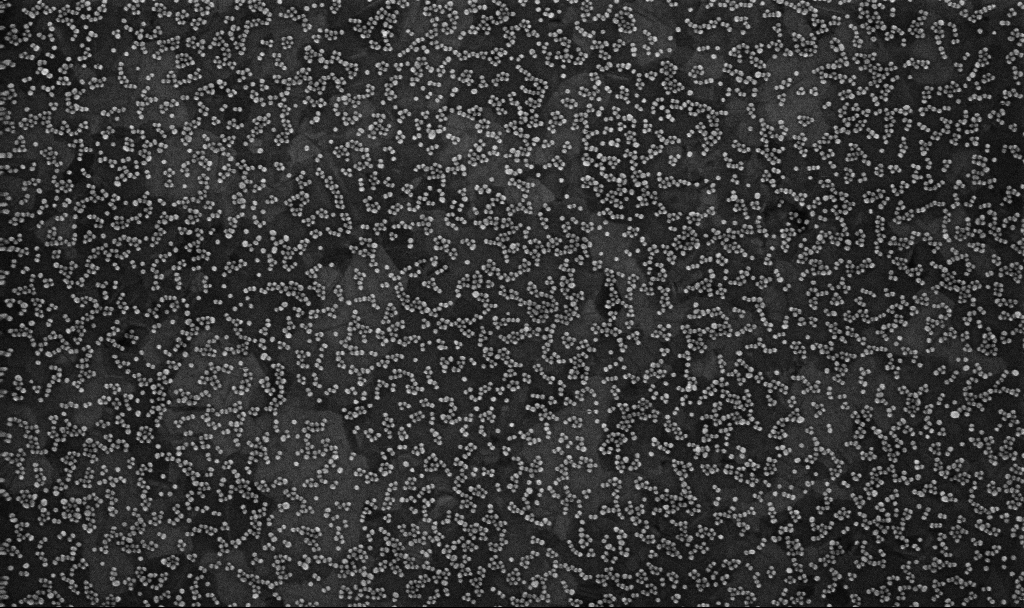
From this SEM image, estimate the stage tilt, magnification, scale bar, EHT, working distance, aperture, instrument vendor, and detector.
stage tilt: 0°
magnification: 100 K X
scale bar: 200 nm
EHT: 10 kV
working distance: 3.3 mm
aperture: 30 µm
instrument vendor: Zeiss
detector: InLens